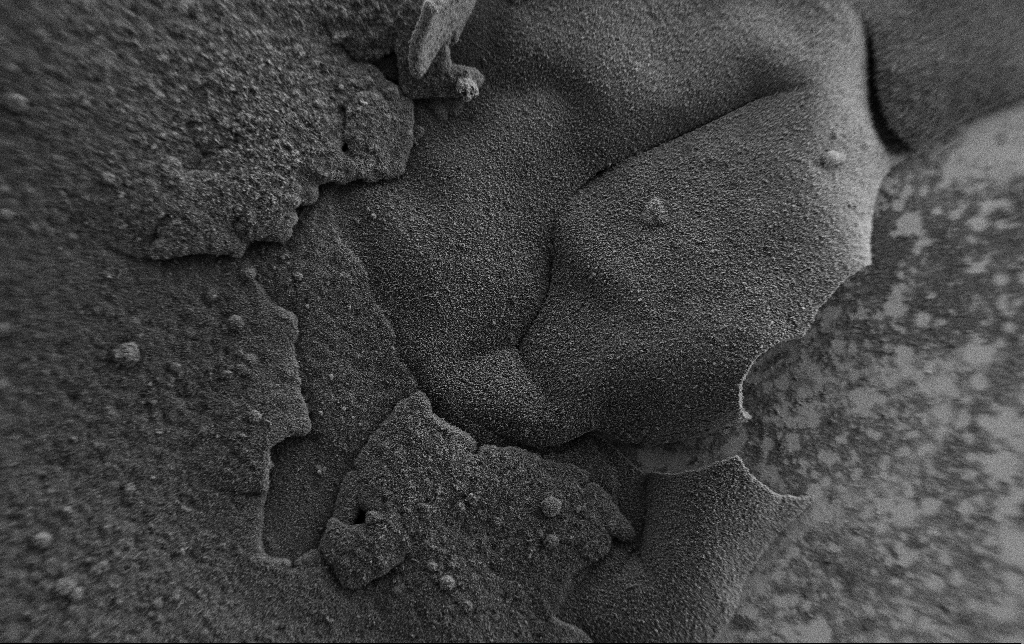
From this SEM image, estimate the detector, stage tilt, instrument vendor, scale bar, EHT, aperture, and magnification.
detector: SE2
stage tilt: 0°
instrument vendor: Zeiss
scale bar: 200000 nm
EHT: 2 kV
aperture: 30 µm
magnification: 0.15 K X